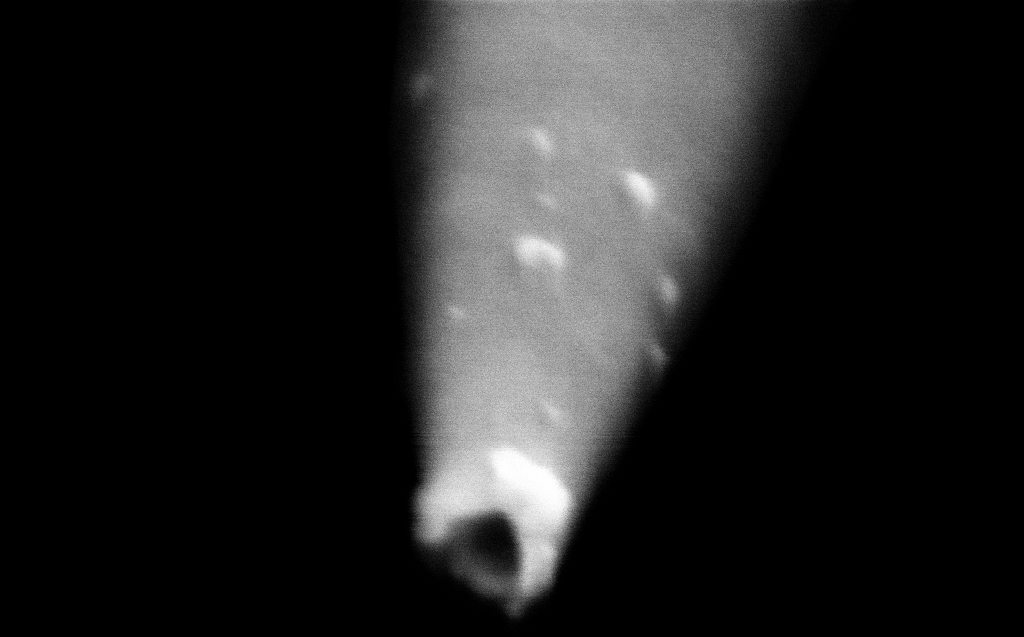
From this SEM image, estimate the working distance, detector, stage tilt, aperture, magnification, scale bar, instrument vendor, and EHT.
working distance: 4 mm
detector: InLens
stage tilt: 45°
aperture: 30 µm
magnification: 500 K X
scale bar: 100 nm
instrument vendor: Zeiss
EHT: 2 kV